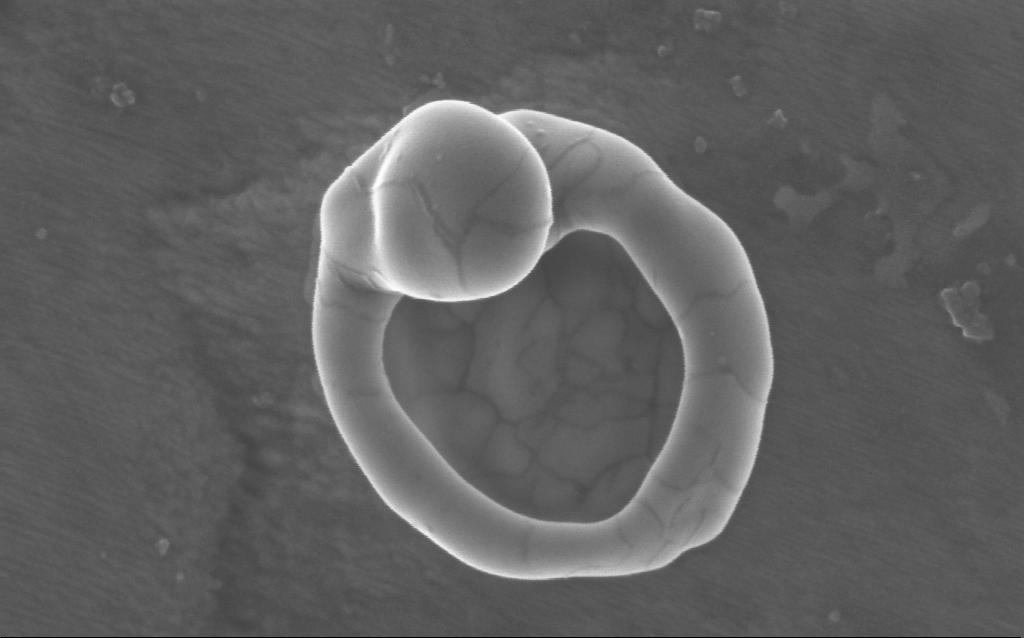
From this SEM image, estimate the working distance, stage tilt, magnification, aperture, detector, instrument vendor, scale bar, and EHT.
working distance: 3 mm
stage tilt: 0°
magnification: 184.18 K X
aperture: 30 µm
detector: InLens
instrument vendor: Zeiss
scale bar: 200 nm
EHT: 5 kV